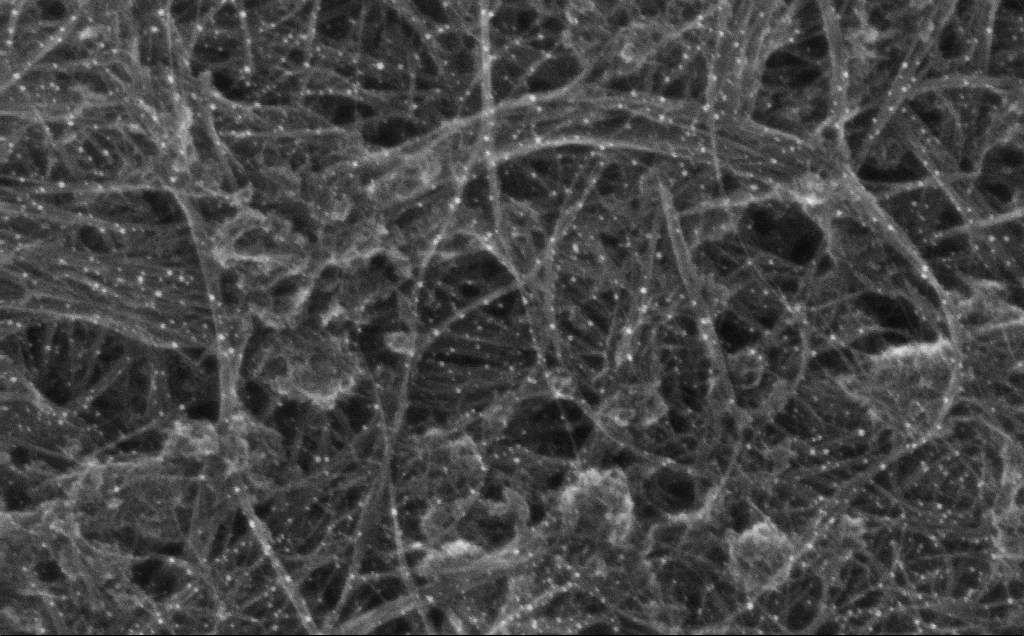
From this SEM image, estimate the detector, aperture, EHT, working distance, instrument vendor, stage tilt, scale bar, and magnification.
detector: InLens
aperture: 30 µm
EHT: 10 kV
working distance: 3 mm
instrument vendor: Zeiss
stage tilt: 0°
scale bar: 200 nm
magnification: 167.14 K X